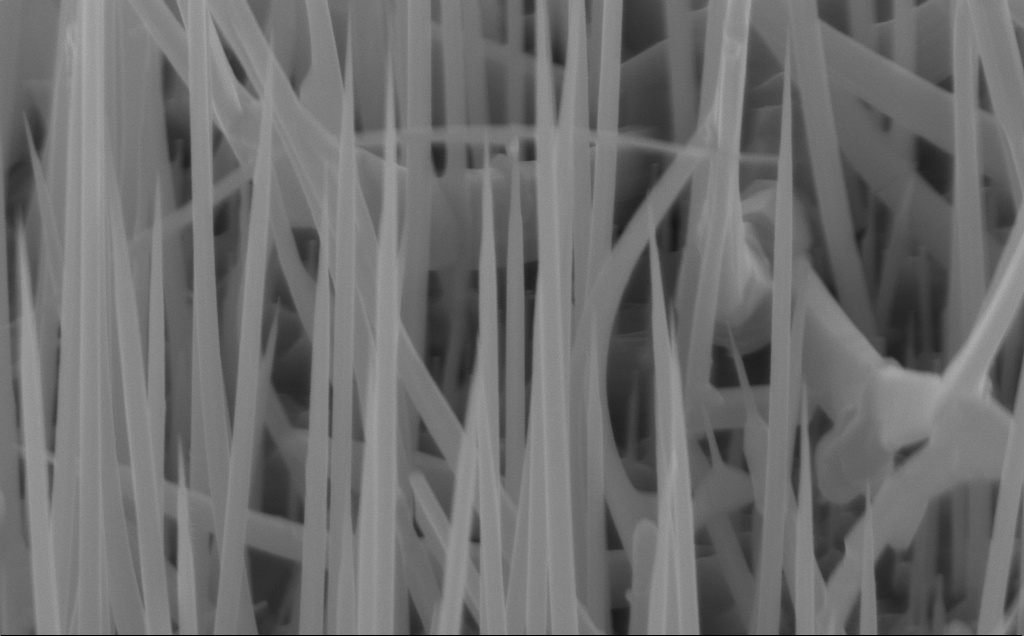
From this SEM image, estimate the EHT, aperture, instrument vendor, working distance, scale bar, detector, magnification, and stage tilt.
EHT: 10 kV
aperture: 30 µm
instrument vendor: Zeiss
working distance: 7 mm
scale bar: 200 nm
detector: InLens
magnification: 72.6 K X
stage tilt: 45°